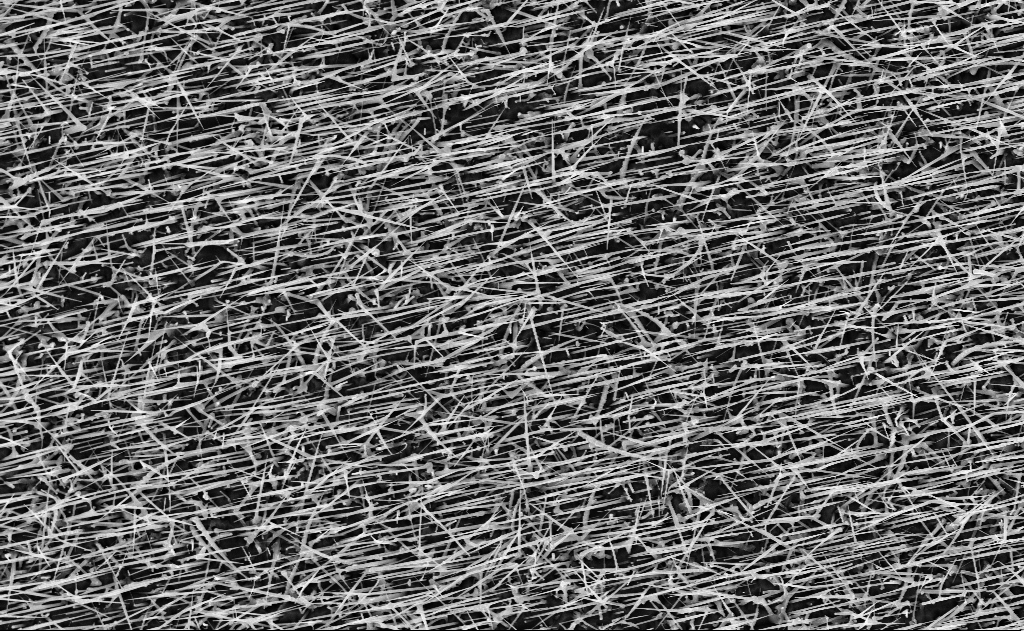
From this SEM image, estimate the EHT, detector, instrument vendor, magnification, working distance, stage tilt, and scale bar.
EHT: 10 kV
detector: InLens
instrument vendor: Zeiss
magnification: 10 K X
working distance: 15 mm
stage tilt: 0°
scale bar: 2000 nm